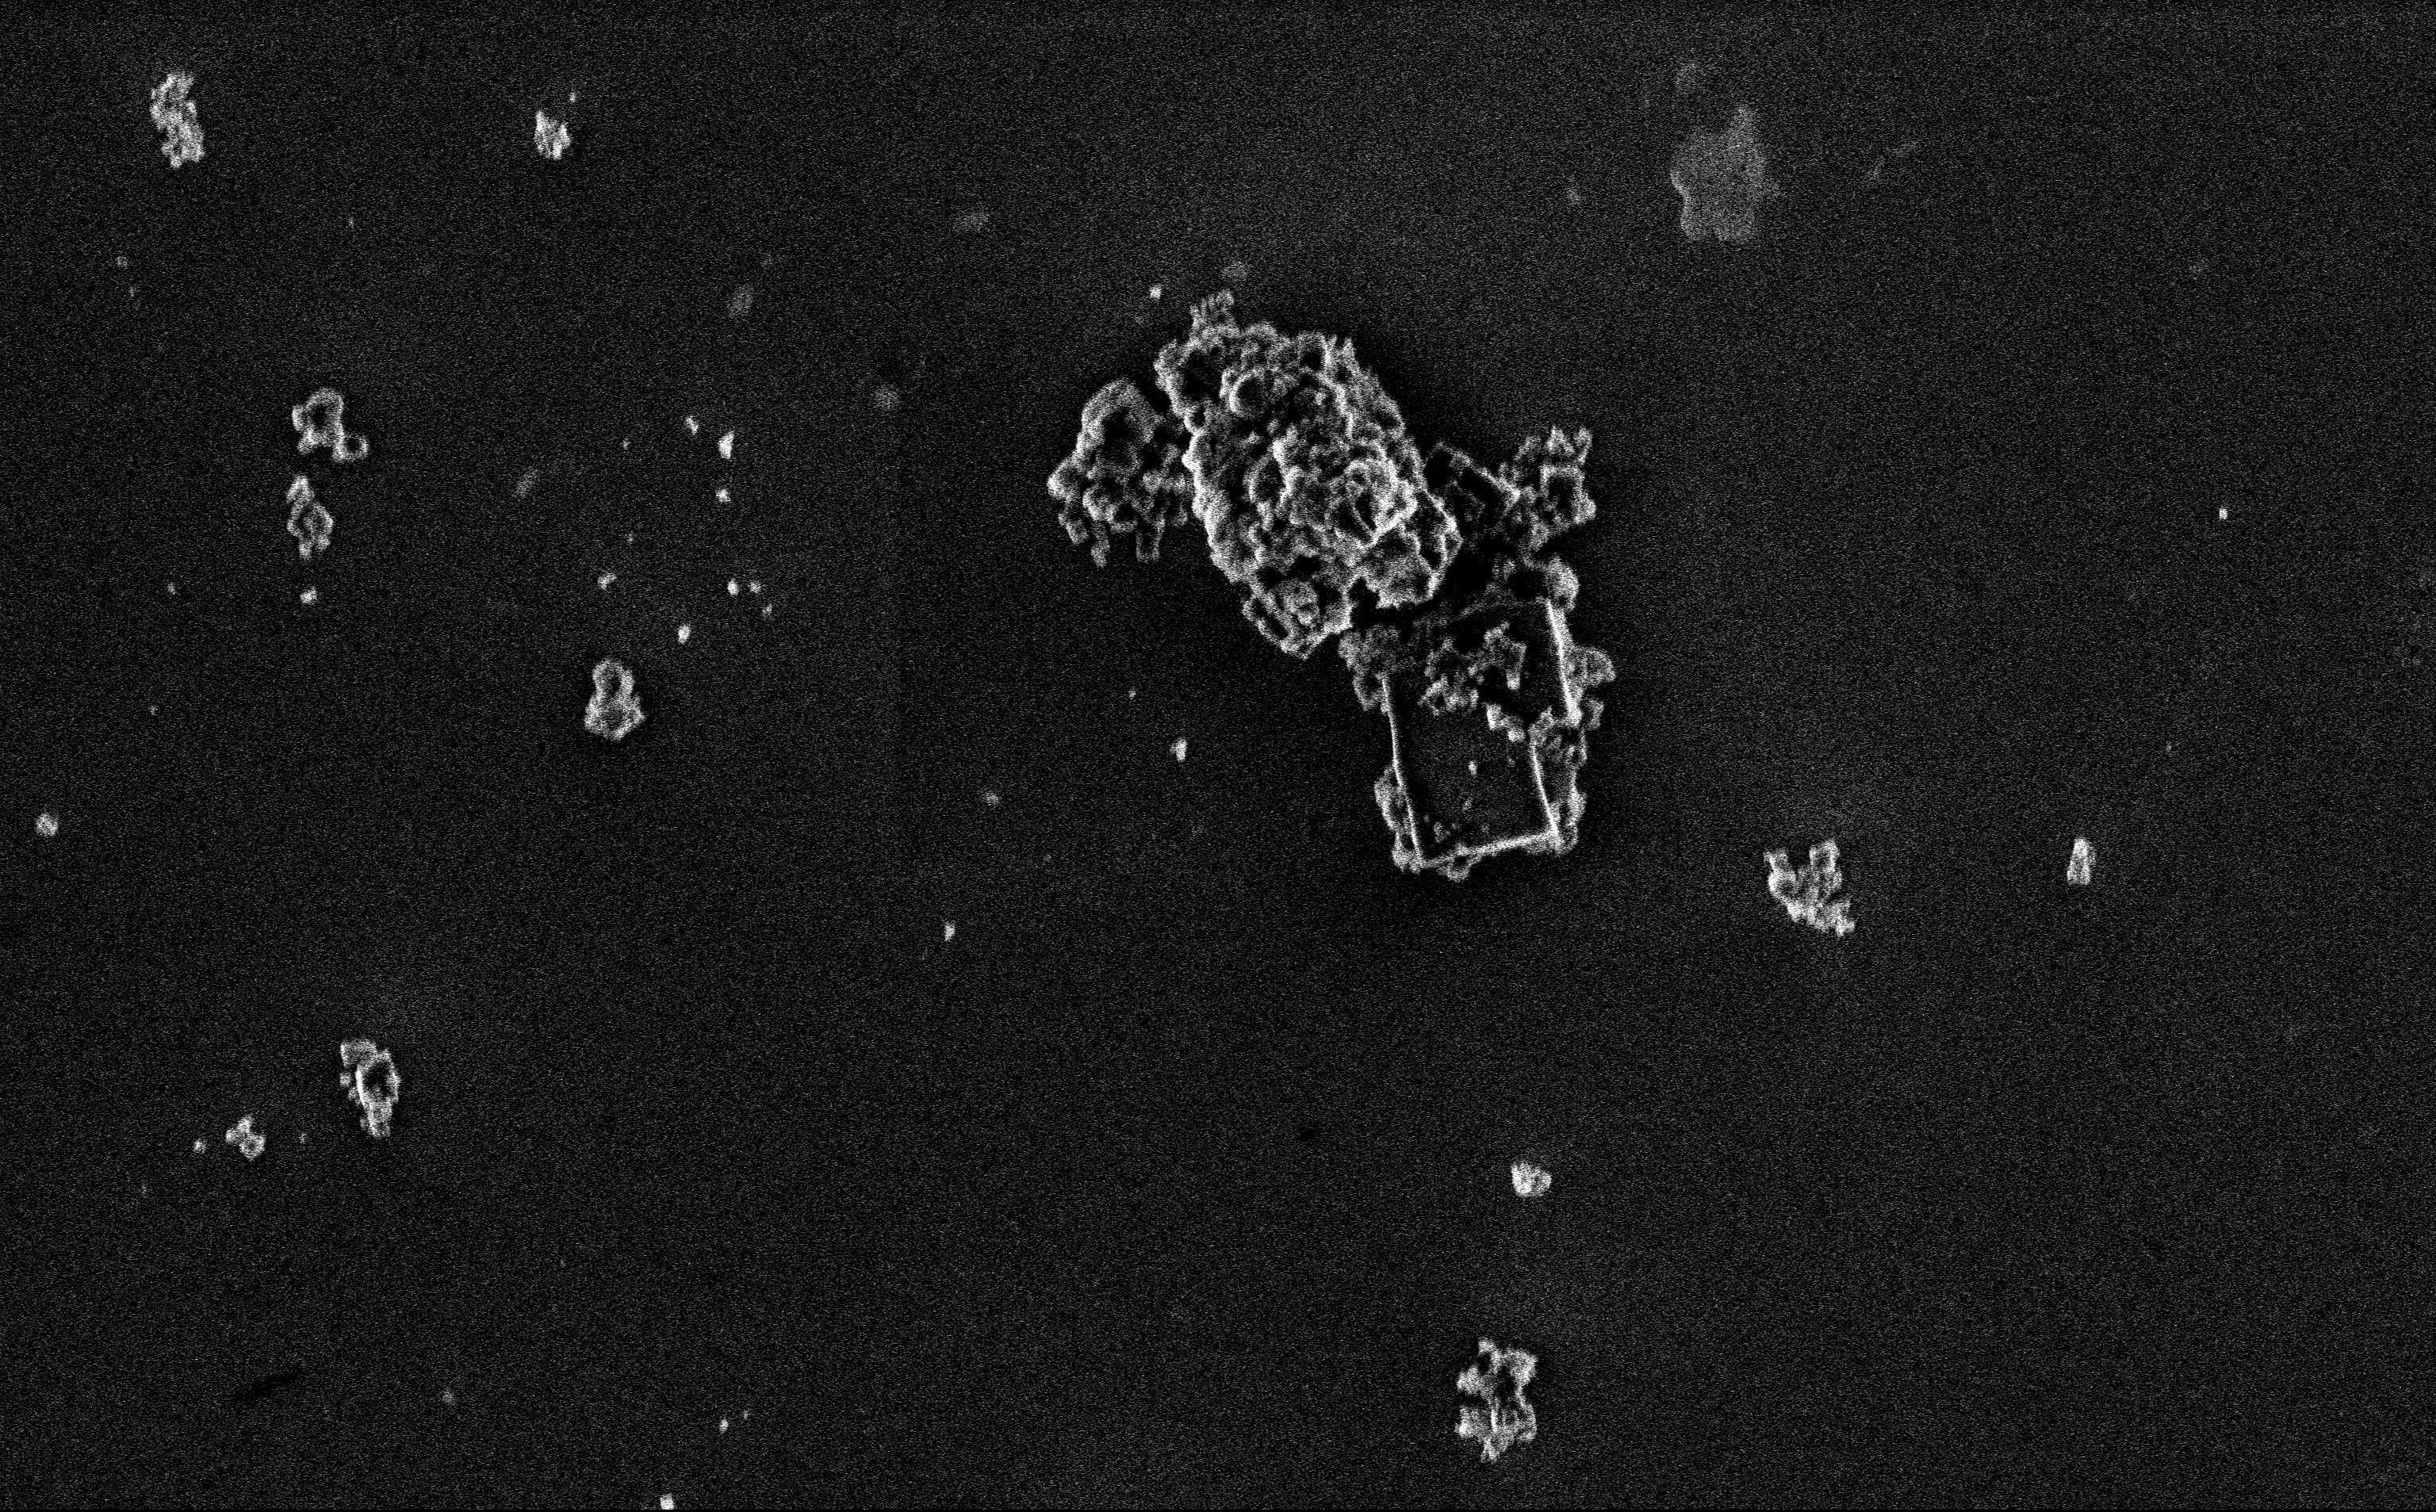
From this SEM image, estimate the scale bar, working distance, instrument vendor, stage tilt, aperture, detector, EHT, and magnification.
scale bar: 2000 nm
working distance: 3 mm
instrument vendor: Zeiss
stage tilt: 0°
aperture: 30 µm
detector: InLens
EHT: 3 kV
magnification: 12.85 K X